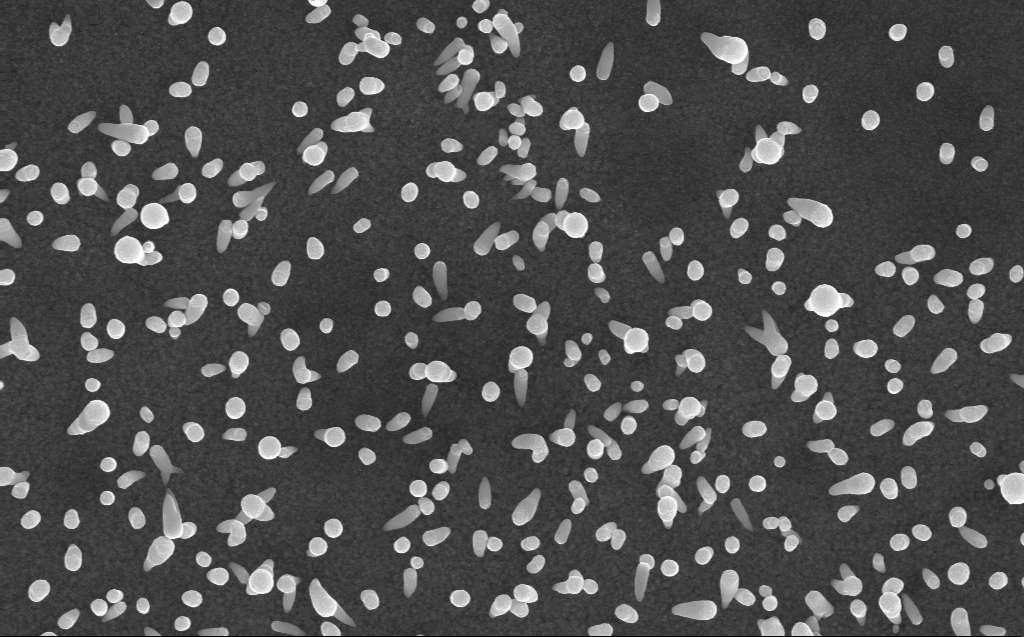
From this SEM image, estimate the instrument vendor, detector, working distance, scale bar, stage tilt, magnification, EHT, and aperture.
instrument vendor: Zeiss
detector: InLens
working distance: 3 mm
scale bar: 1000 nm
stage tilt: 0°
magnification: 50 K X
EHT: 10 kV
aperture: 30 µm